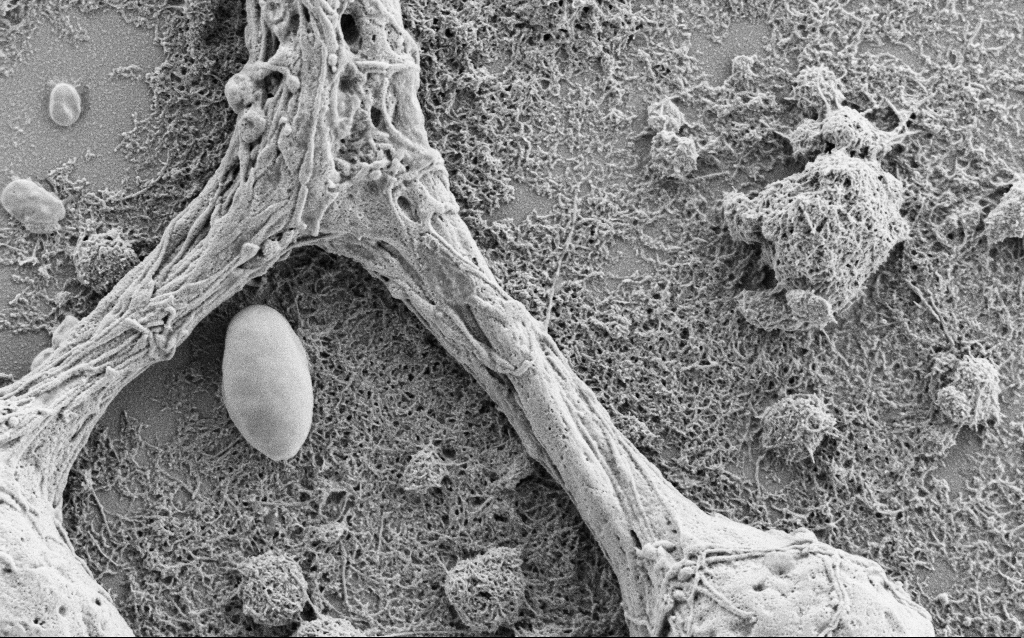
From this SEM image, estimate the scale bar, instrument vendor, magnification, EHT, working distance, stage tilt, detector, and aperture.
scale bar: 1000 nm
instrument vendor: Zeiss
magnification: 15 K X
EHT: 1.5 kV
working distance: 6.9 mm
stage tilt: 0°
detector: SE2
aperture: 30 µm